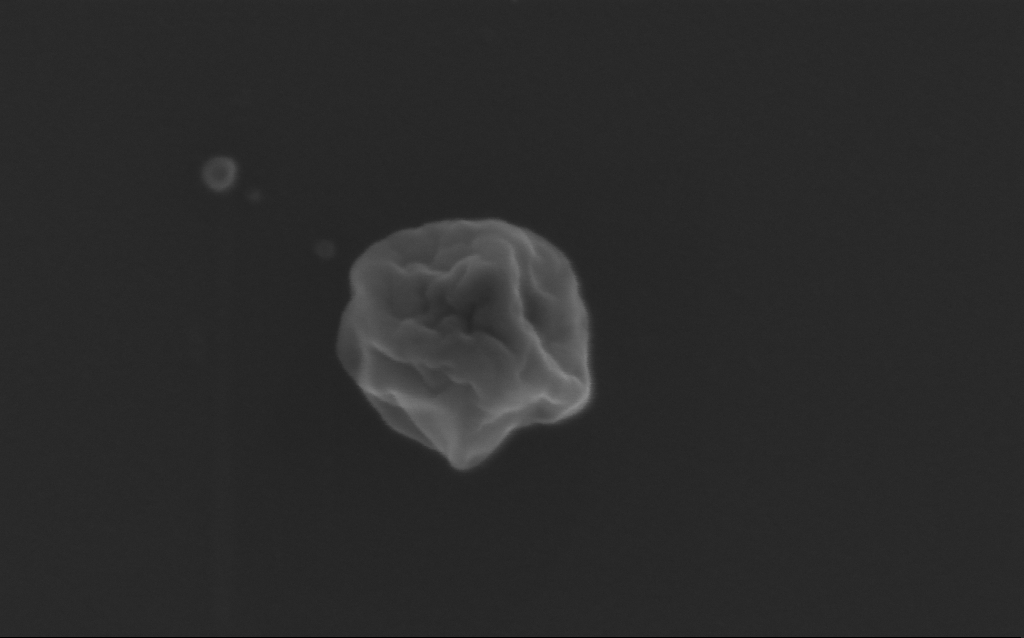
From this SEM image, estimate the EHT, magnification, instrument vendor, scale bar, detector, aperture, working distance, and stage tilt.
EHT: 10 kV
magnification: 403.29 K X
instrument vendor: Zeiss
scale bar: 100 nm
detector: InLens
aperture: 30 µm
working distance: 3 mm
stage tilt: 0°